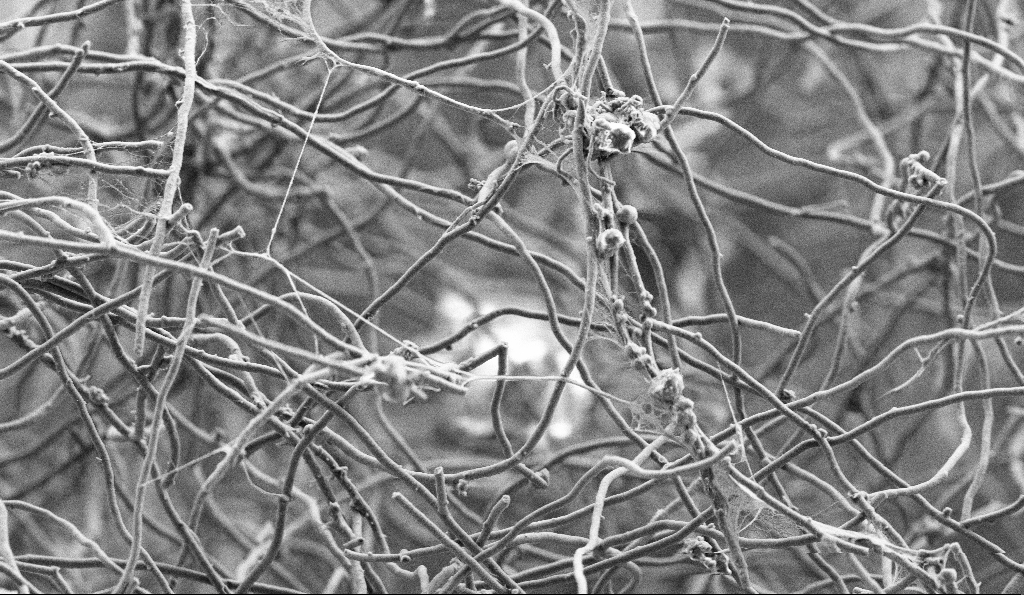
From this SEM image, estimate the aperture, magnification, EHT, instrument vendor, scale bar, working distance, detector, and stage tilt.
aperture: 30 µm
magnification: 5 K X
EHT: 3 kV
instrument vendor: Zeiss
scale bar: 10000 nm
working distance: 5 mm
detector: SE2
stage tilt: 0°